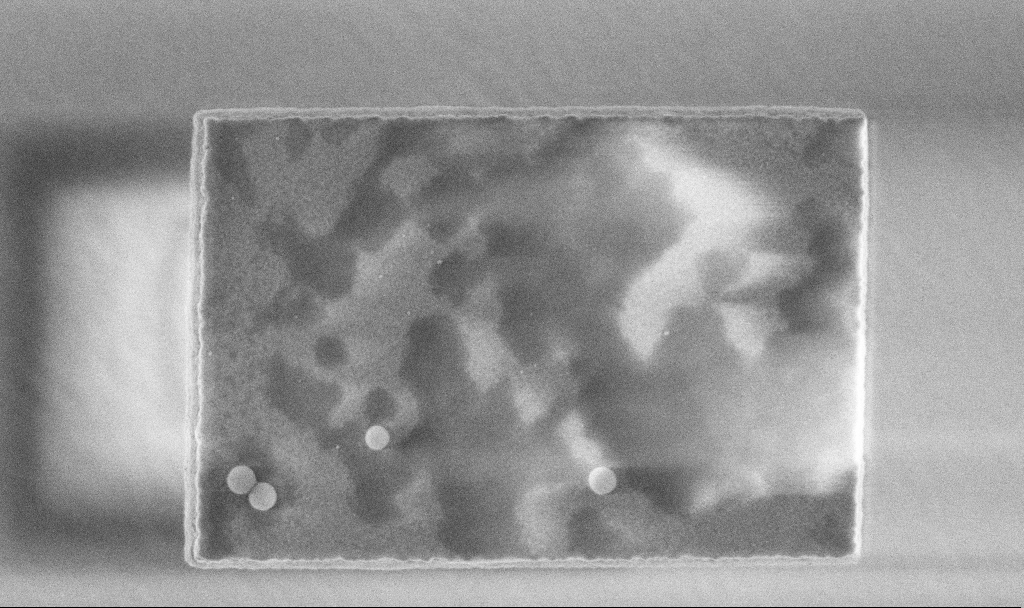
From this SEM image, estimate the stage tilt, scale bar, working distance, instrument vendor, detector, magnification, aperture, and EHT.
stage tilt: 0°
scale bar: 1000 nm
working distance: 3.3 mm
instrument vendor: Zeiss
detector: InLens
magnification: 55.48 K X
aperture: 30 µm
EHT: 3 kV